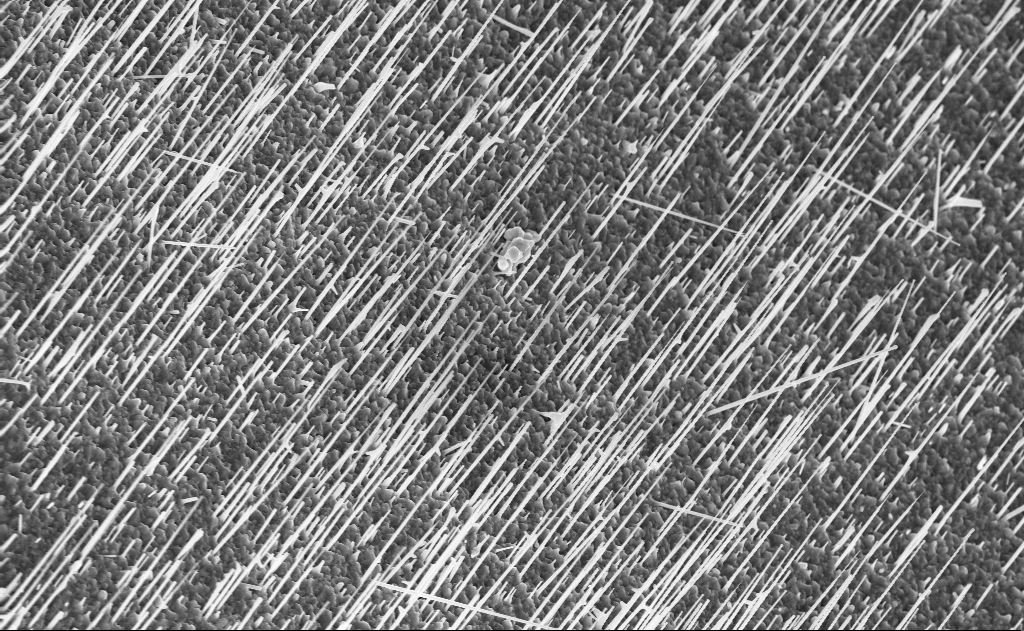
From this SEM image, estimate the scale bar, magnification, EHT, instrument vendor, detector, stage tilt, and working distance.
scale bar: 2000 nm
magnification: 10 K X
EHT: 10 kV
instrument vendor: Zeiss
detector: InLens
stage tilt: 0°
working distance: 10 mm